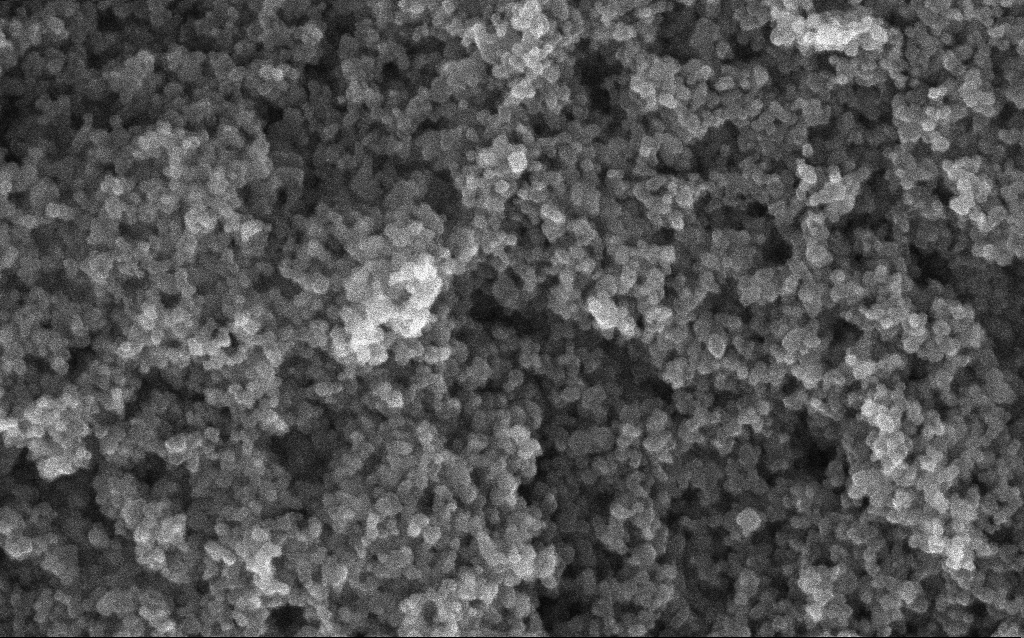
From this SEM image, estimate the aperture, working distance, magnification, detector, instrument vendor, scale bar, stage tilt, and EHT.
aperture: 30 µm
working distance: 2.8 mm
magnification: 204.13 K X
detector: InLens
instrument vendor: Zeiss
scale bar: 100 nm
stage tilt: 0°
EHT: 10 kV